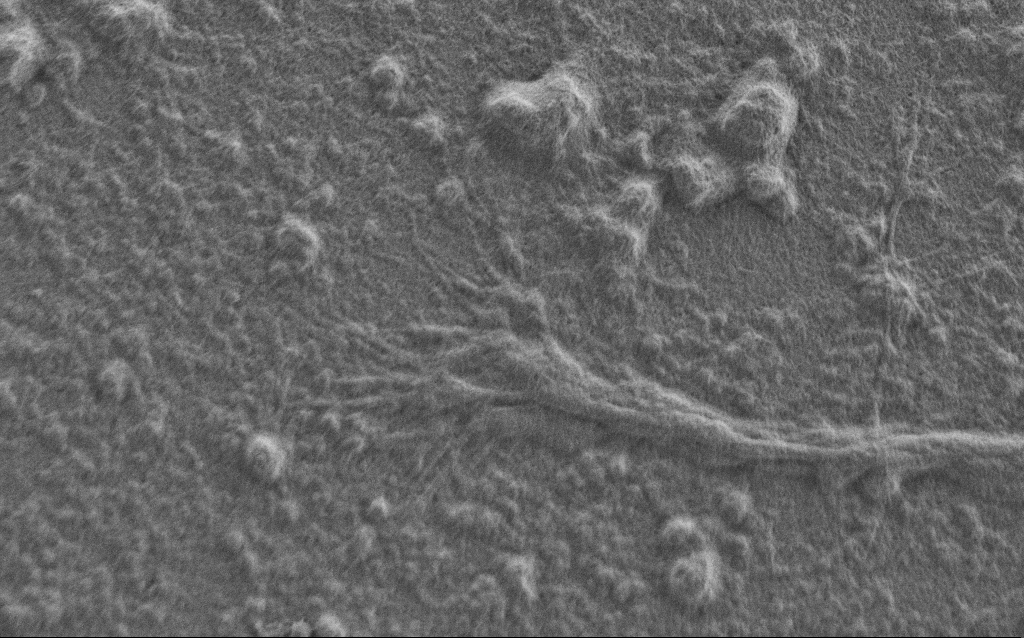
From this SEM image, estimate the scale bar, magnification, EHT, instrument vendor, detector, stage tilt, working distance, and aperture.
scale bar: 2000 nm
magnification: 7.5 K X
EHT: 0.9 kV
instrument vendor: Zeiss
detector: SE2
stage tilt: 0°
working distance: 7 mm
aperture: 30 µm